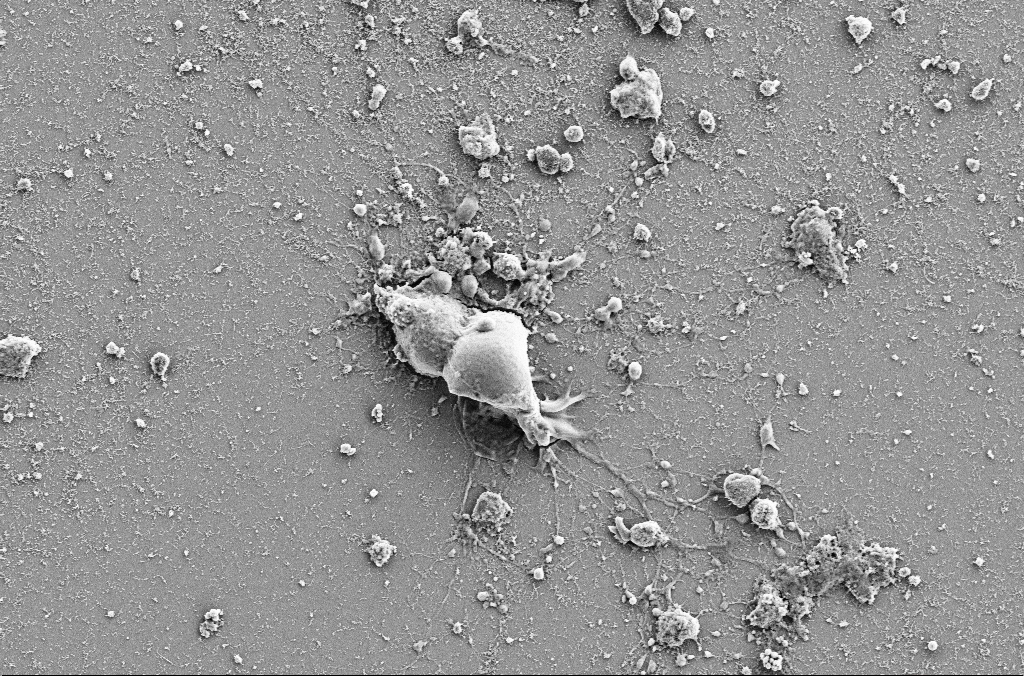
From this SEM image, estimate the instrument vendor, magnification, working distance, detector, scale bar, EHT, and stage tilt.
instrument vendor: Zeiss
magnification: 3 K X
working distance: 4 mm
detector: SE2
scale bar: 10000 nm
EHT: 5 kV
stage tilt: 0°